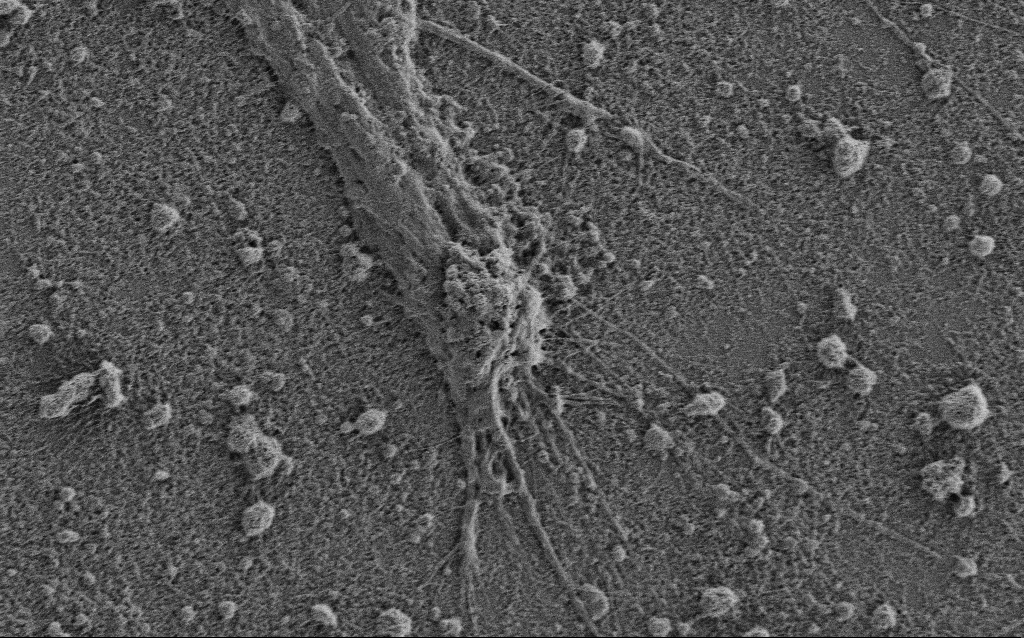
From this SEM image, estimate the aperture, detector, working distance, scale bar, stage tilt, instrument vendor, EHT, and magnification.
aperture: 30 µm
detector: SE2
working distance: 3.4 mm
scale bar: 10000 nm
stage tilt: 0°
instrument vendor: Zeiss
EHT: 0.9 kV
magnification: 5 K X